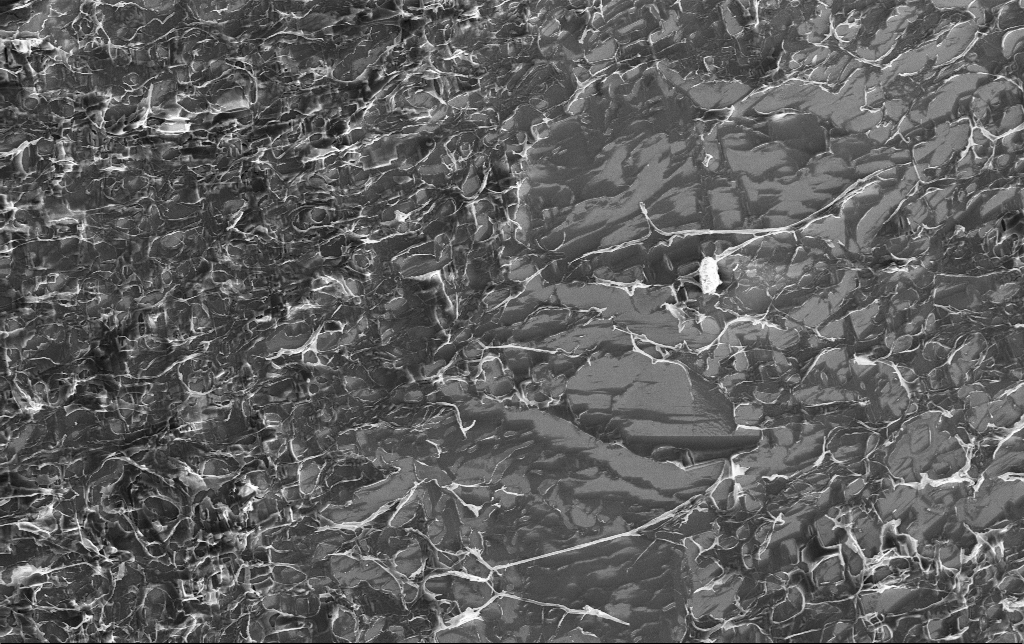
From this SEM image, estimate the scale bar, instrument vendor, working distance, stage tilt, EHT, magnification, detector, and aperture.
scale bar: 10000 nm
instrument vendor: Zeiss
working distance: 3.2 mm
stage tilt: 0°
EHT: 3 kV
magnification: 3.27 K X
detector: InLens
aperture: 30 µm